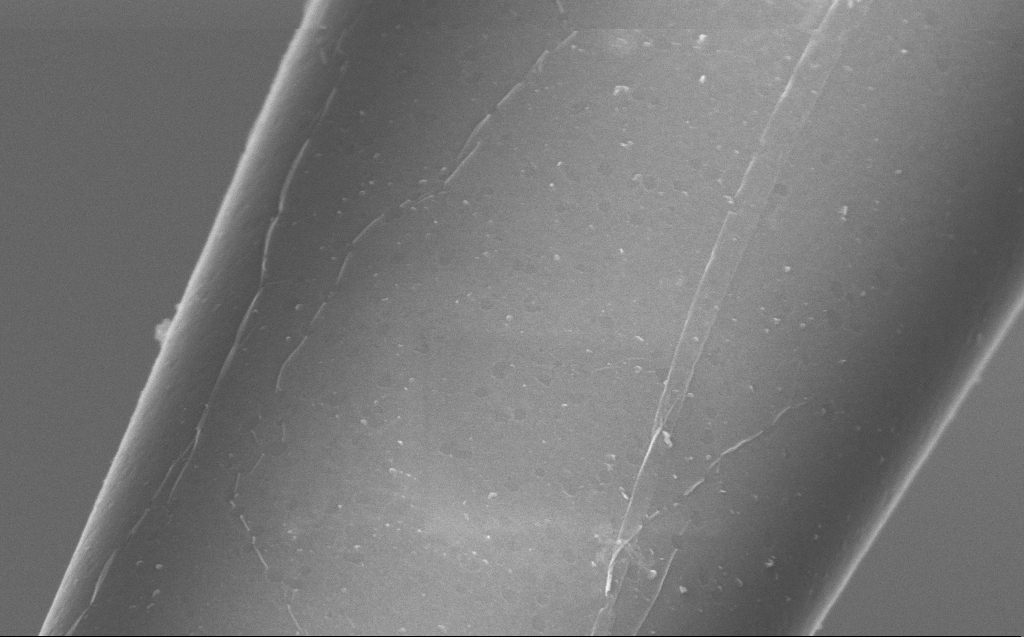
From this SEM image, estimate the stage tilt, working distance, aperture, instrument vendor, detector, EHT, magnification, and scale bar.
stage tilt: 45°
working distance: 6 mm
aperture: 30 µm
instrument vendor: Zeiss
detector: InLens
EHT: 5 kV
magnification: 100 K X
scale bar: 200 nm